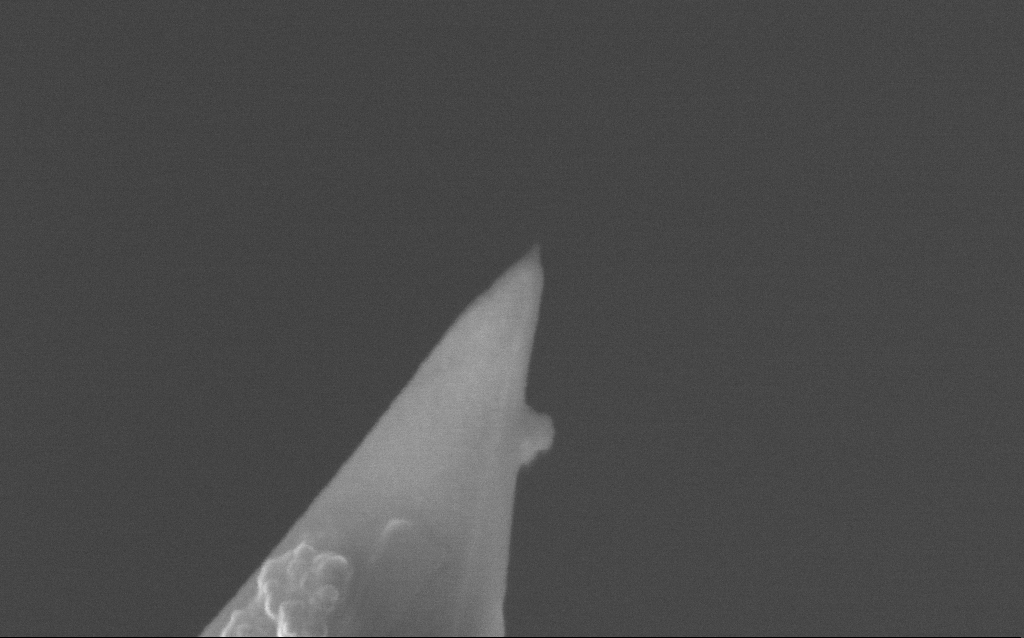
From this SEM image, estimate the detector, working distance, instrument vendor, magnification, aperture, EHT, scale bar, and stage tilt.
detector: InLens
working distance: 4.7 mm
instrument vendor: Zeiss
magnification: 250 K X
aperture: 30 µm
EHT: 5 kV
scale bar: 100 nm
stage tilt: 0°